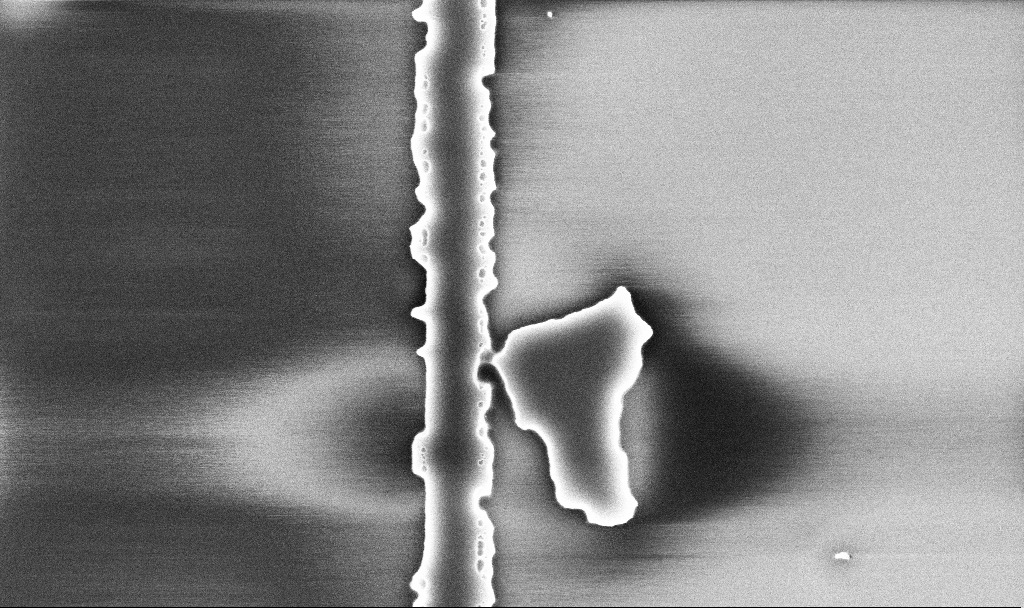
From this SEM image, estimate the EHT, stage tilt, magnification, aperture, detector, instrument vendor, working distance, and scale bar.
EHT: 5 kV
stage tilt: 0°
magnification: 38.7 K X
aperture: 30 µm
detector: InLens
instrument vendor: Zeiss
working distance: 10.1 mm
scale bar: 1000 nm